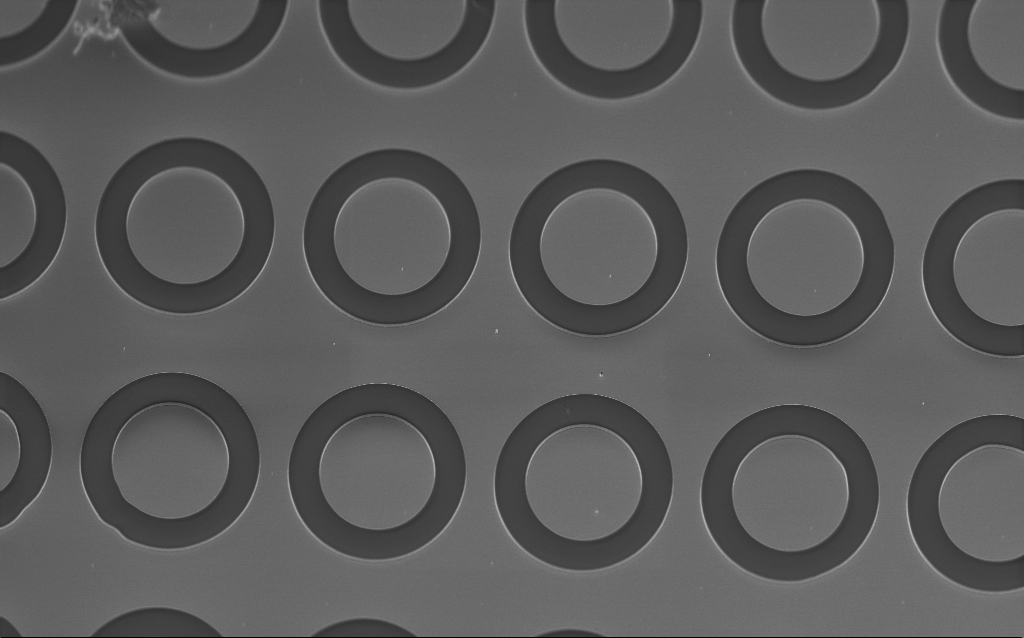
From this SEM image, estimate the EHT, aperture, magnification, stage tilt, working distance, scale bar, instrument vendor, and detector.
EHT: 2 kV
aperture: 30 µm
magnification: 1.1 K X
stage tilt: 45°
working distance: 3.4 mm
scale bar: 20000 nm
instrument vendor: Zeiss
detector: InLens